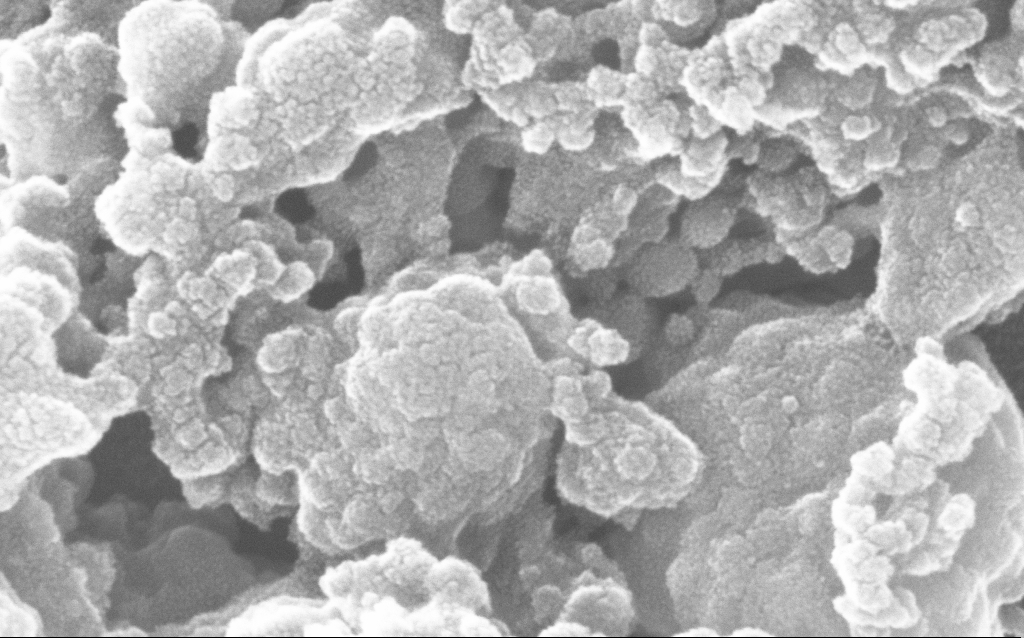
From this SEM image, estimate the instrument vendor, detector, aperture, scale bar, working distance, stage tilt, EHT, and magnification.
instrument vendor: Zeiss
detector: InLens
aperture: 30 µm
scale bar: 100 nm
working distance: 1.7 mm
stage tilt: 0°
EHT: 20 kV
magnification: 500 K X